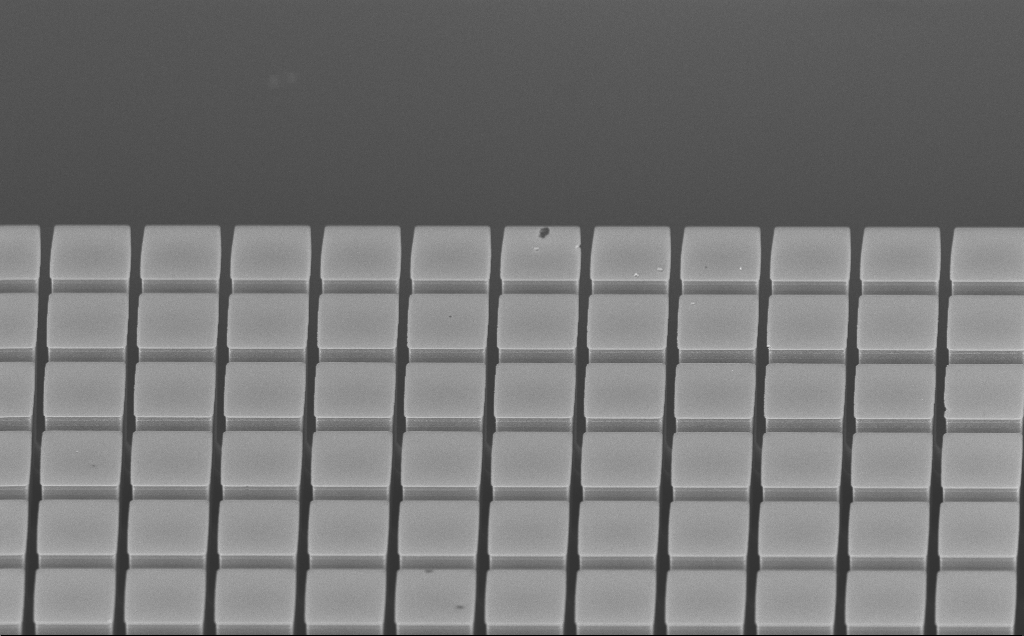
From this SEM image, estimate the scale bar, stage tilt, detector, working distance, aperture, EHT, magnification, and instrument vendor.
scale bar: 10000 nm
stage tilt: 60°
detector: InLens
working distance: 7 mm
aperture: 30 µm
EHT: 20 kV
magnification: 6.6 K X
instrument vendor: Zeiss